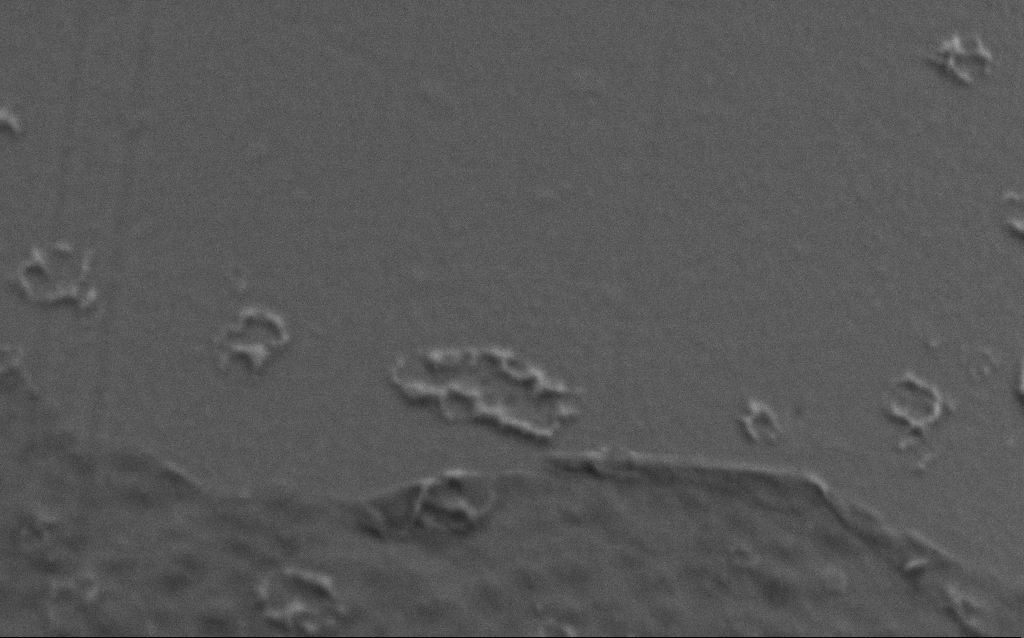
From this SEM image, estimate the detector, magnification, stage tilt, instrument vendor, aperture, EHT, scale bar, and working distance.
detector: SE2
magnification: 100 K X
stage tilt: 45°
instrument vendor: Zeiss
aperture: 30 µm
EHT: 1 kV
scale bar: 200 nm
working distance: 6 mm